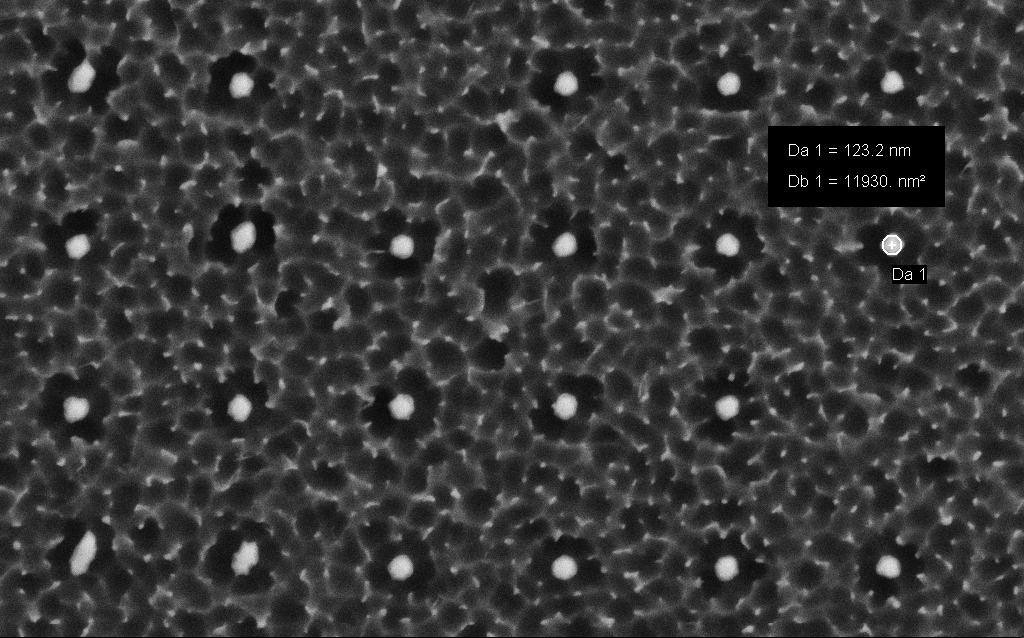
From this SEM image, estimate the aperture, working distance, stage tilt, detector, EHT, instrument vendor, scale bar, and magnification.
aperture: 30 µm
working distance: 5 mm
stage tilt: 0°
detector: SE2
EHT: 3 kV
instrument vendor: Zeiss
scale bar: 1000 nm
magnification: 59.58 K X